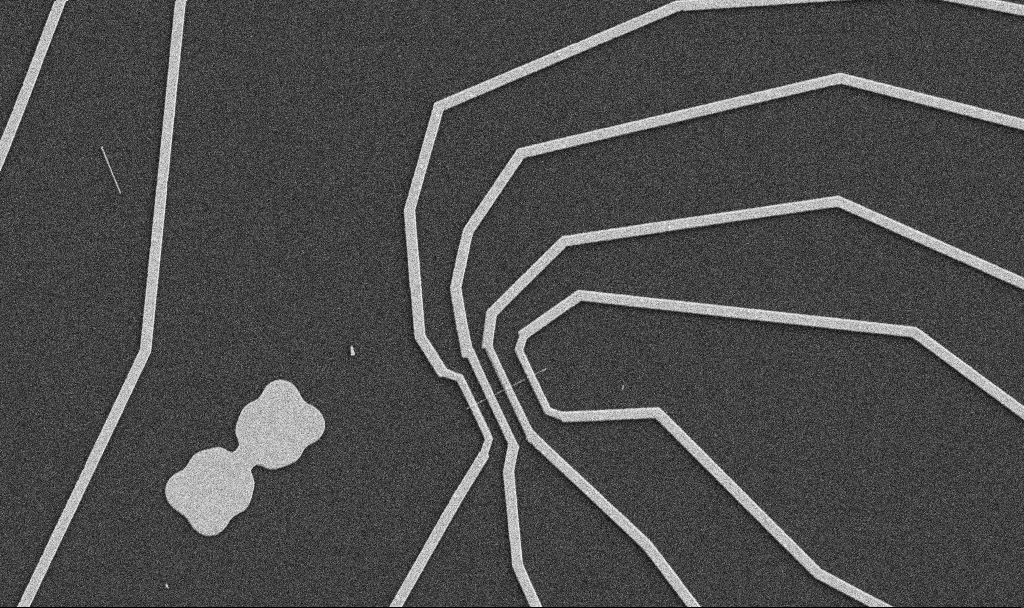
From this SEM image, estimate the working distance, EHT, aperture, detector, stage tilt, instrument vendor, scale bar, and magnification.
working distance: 10.6 mm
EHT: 5 kV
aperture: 30 µm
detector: SE2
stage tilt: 0°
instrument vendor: Zeiss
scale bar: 10000 nm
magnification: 5 K X